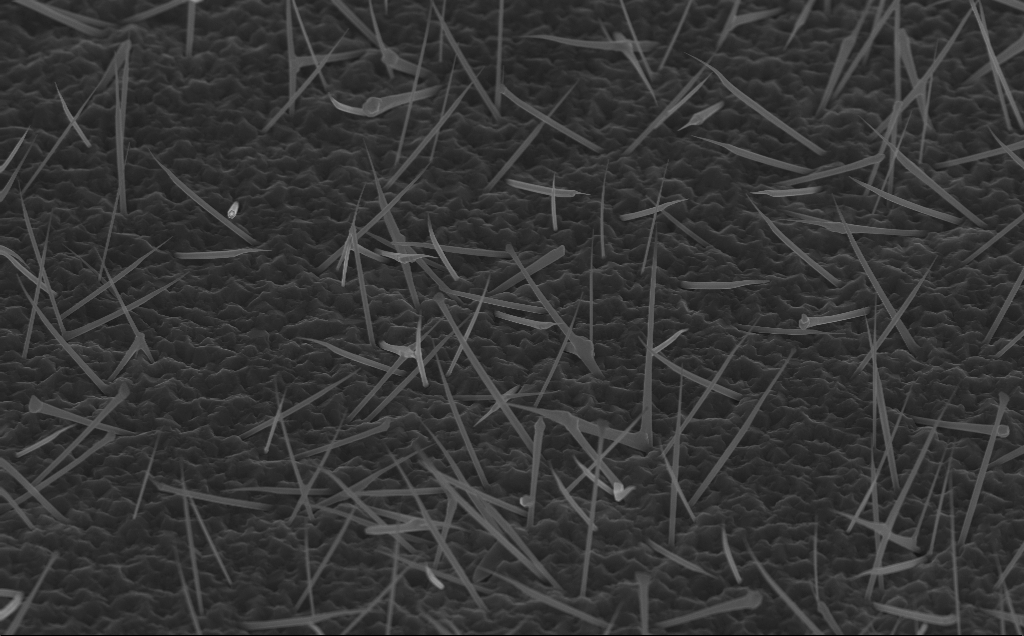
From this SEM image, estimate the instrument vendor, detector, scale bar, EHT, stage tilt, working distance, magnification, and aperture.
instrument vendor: Zeiss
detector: InLens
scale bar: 2000 nm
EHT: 10 kV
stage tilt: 45°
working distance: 5 mm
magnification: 20 K X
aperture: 30 µm